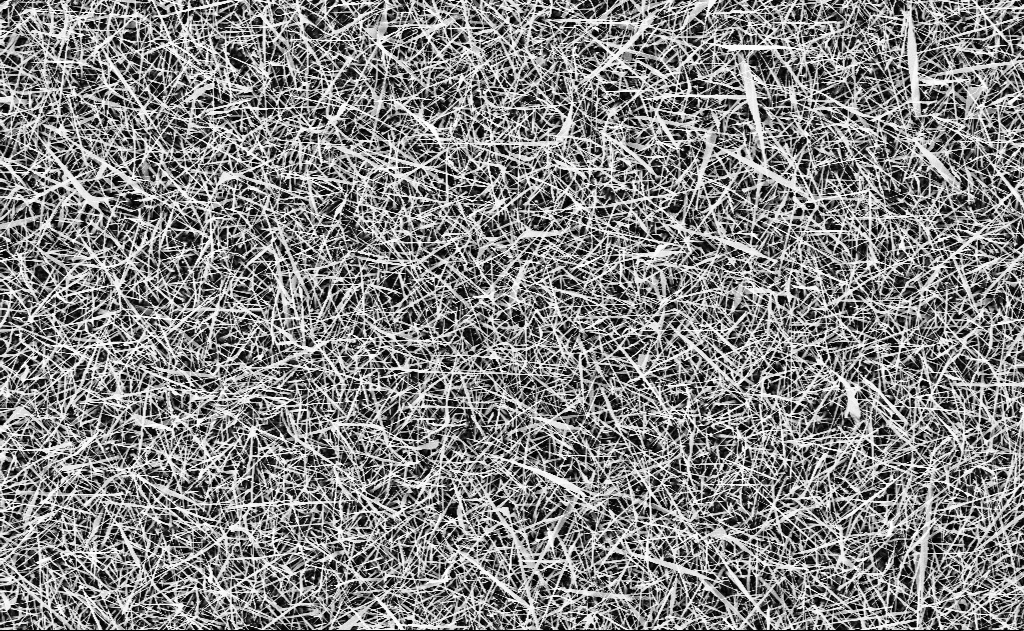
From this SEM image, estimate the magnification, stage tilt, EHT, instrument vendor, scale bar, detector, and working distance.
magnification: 5 K X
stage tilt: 0°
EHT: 10 kV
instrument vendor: Zeiss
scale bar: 10000 nm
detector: InLens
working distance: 15 mm